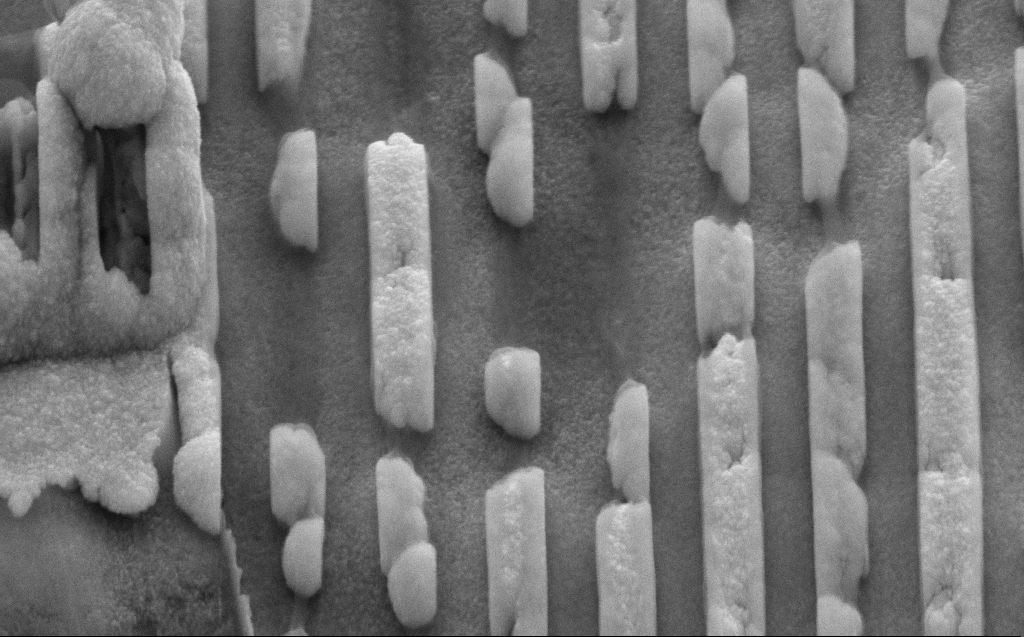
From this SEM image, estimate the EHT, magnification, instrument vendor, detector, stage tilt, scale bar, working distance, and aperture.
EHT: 10 kV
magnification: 80.41 K X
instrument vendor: Zeiss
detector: SE2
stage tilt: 45°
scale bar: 200 nm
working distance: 8 mm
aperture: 30 µm